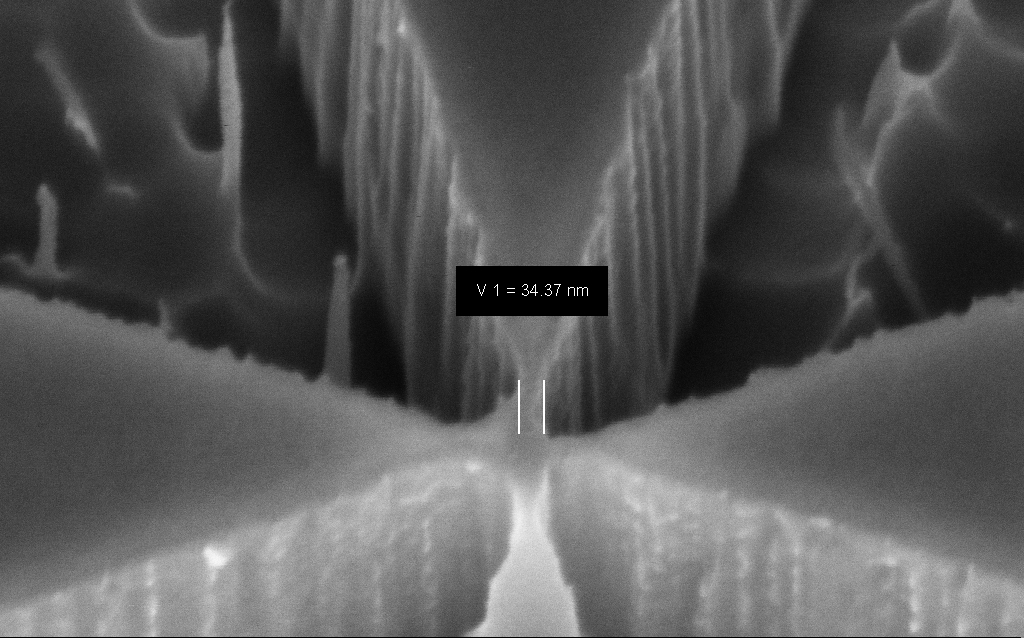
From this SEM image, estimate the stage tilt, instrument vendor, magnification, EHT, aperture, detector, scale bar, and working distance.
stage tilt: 45°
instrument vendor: Zeiss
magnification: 267.05 K X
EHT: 3 kV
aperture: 30 µm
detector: InLens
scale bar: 100 nm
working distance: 8 mm